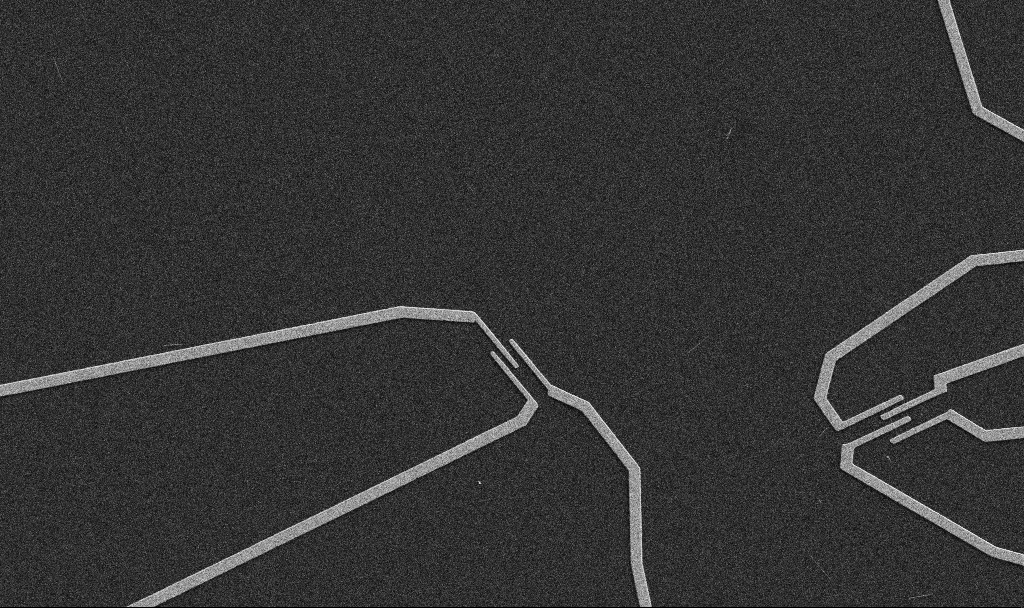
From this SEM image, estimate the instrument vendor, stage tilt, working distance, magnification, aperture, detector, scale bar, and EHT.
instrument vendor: Zeiss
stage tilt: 0°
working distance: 10.7 mm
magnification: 5 K X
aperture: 30 µm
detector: SE2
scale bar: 10000 nm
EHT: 5 kV